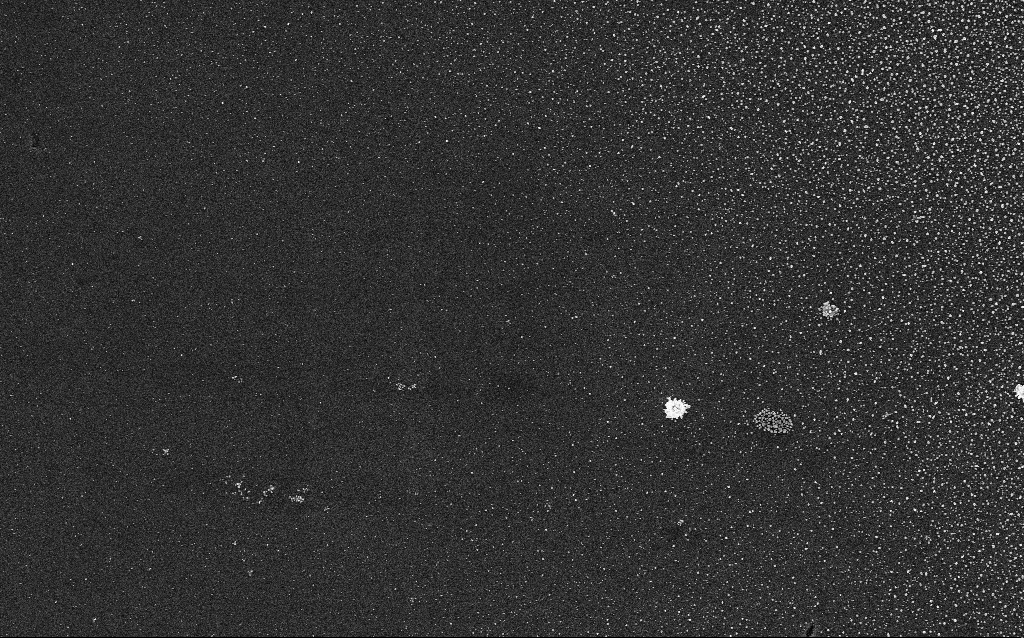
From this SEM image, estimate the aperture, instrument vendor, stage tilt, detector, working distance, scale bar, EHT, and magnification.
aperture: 30 µm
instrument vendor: Zeiss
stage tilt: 0°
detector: InLens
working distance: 2.1 mm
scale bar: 2000 nm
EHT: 5 kV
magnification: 10 K X